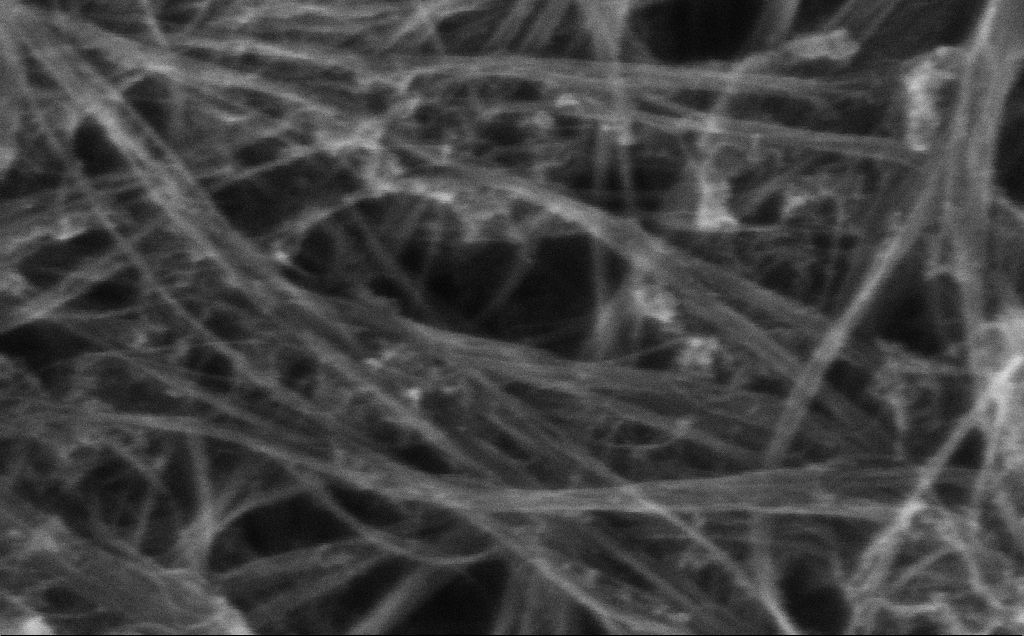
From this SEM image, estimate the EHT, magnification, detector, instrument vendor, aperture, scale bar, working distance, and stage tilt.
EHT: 10 kV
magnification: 325.16 K X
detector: InLens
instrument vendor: Zeiss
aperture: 30 µm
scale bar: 200 nm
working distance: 3 mm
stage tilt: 0°